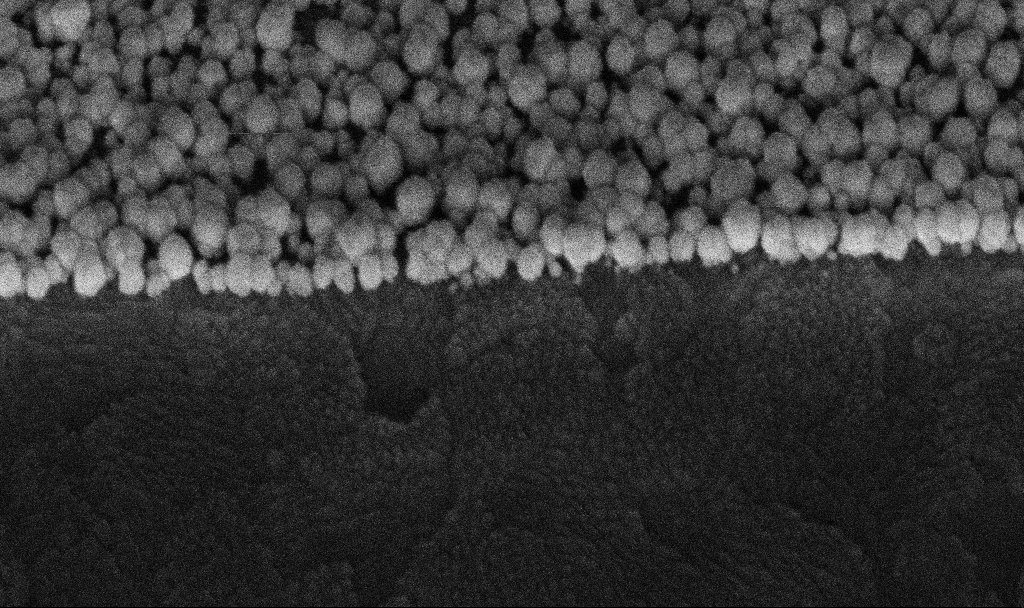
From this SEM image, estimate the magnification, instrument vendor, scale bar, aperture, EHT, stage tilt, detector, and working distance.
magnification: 186.24 K X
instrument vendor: Zeiss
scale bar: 200 nm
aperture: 30 µm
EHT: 5 kV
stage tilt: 45°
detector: InLens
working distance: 6.4 mm